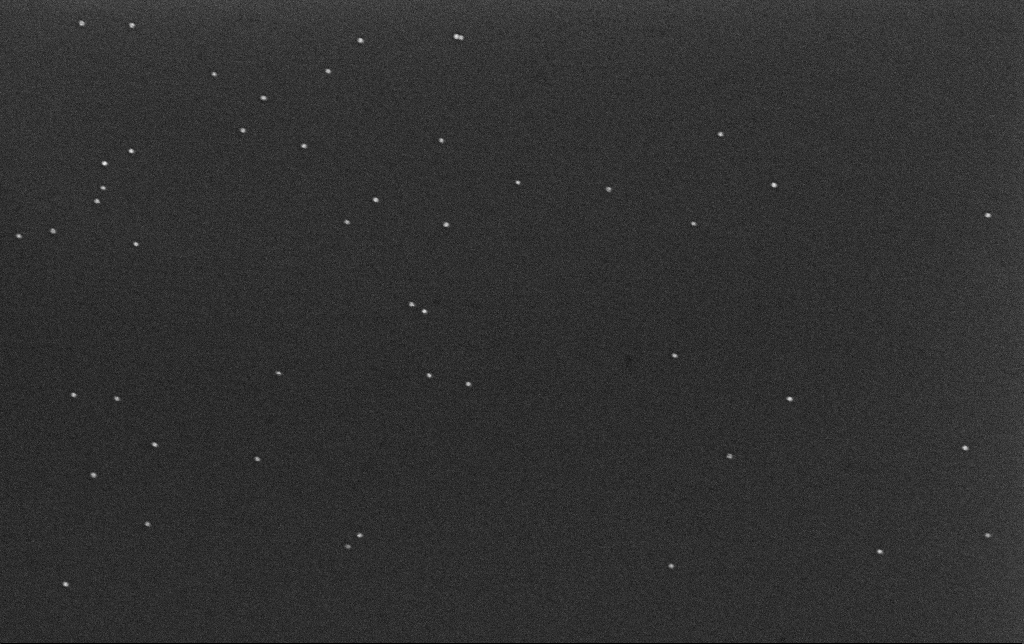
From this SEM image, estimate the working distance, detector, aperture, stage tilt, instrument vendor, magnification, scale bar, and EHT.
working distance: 3.2 mm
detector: InLens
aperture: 30 µm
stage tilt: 0°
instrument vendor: Zeiss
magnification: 100 K X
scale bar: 200 nm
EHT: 10 kV